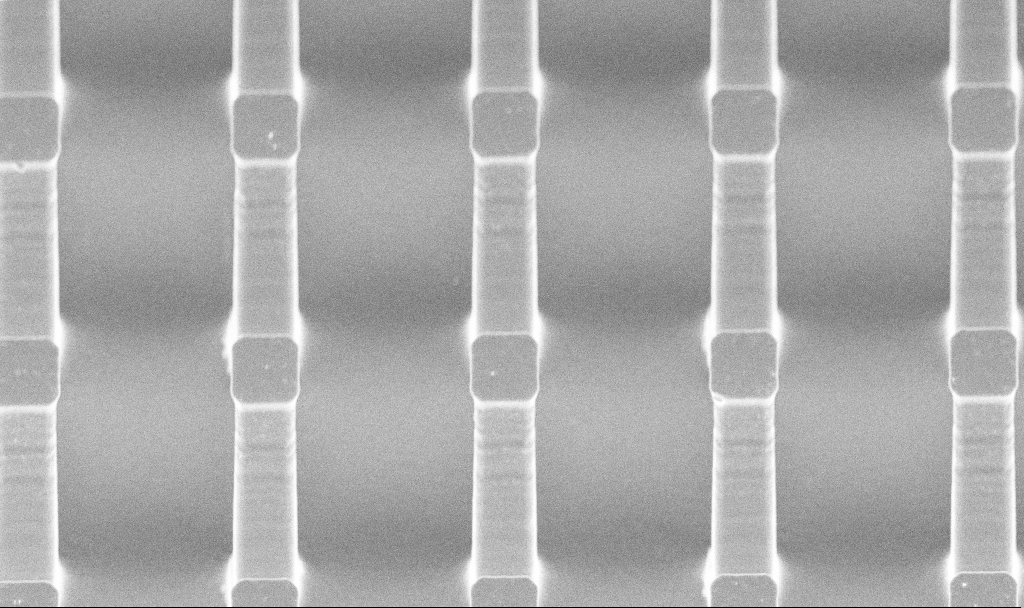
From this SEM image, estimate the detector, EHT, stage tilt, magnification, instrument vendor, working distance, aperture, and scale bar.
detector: InLens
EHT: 5 kV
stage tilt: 45°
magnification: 2.94 K X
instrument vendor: Zeiss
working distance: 10 mm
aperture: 30 µm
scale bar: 20000 nm